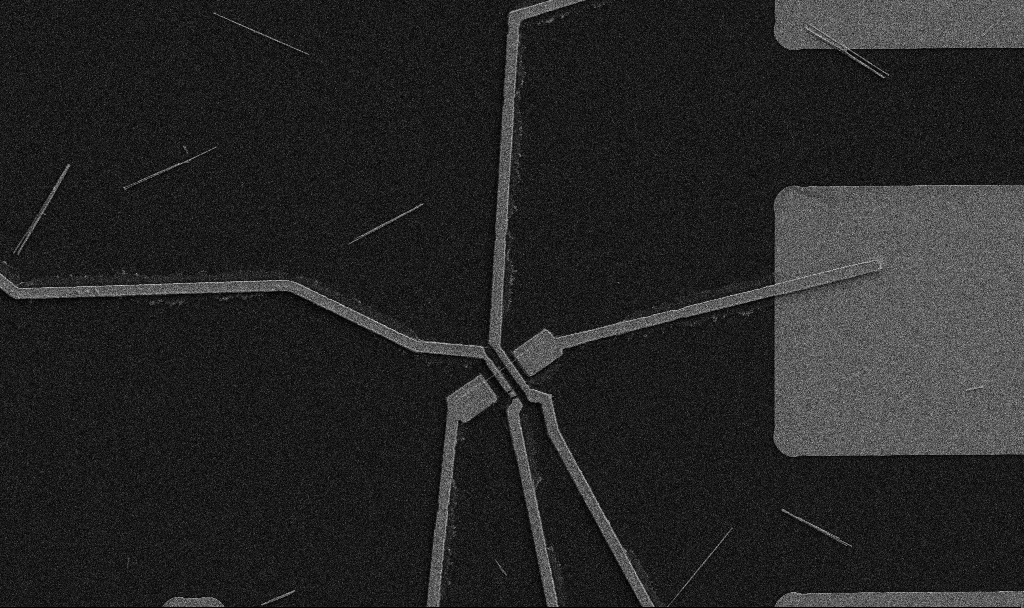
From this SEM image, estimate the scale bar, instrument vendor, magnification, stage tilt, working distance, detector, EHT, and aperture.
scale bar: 10000 nm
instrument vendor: Zeiss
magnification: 5 K X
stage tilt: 0°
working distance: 10.7 mm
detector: SE2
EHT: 5 kV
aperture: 30 µm